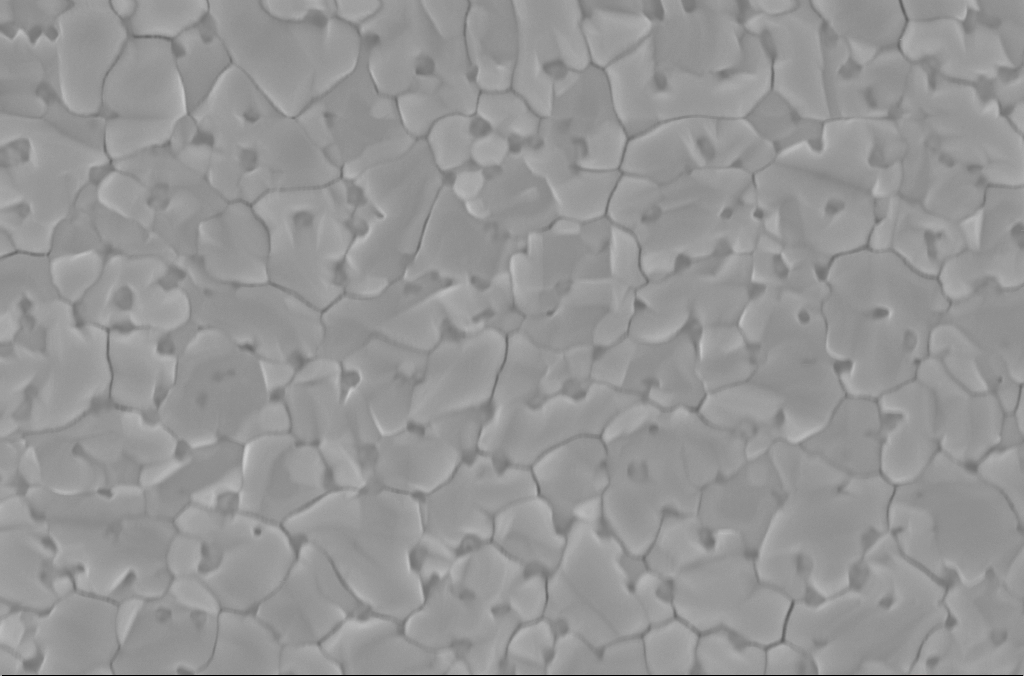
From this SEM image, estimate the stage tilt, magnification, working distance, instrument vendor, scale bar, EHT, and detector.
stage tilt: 0°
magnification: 50 K X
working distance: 3 mm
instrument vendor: Zeiss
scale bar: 1000 nm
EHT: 5 kV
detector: InLens